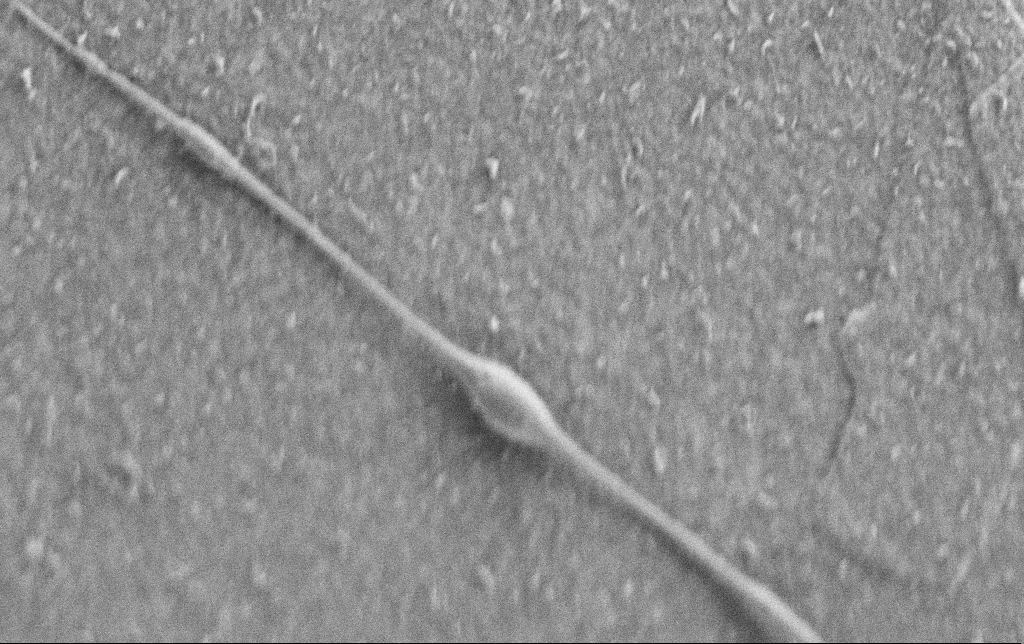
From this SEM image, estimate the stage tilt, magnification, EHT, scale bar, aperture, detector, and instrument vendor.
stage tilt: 0°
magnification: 20 K X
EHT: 0.9 kV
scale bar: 2000 nm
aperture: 30 µm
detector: SE2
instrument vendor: Zeiss